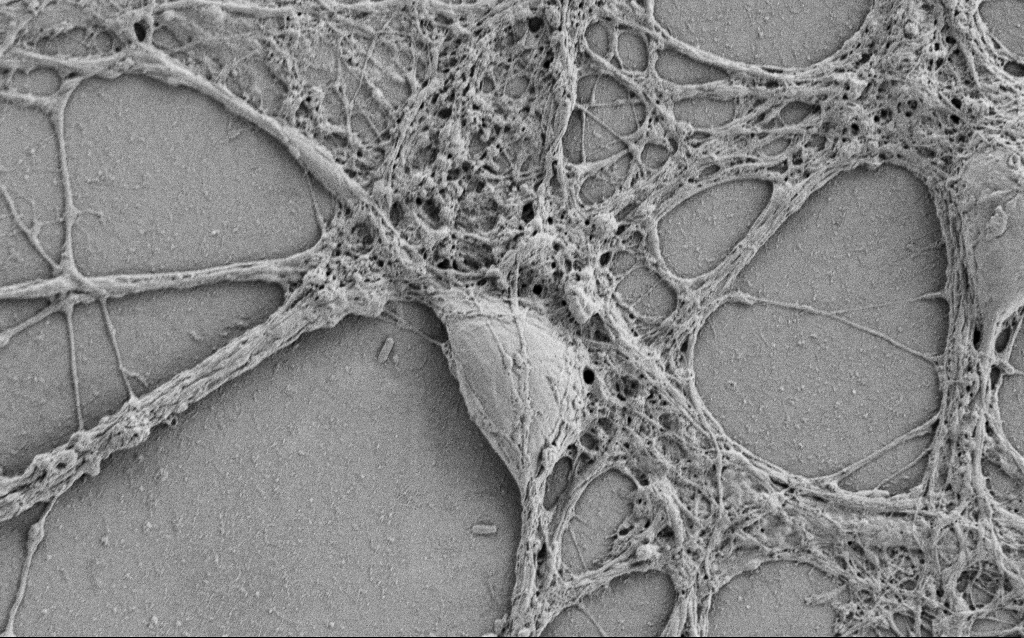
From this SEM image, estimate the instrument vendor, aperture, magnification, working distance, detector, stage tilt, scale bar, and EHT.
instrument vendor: Zeiss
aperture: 30 µm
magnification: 5 K X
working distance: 5.7 mm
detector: SE2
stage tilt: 0°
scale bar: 10000 nm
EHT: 0.8 kV